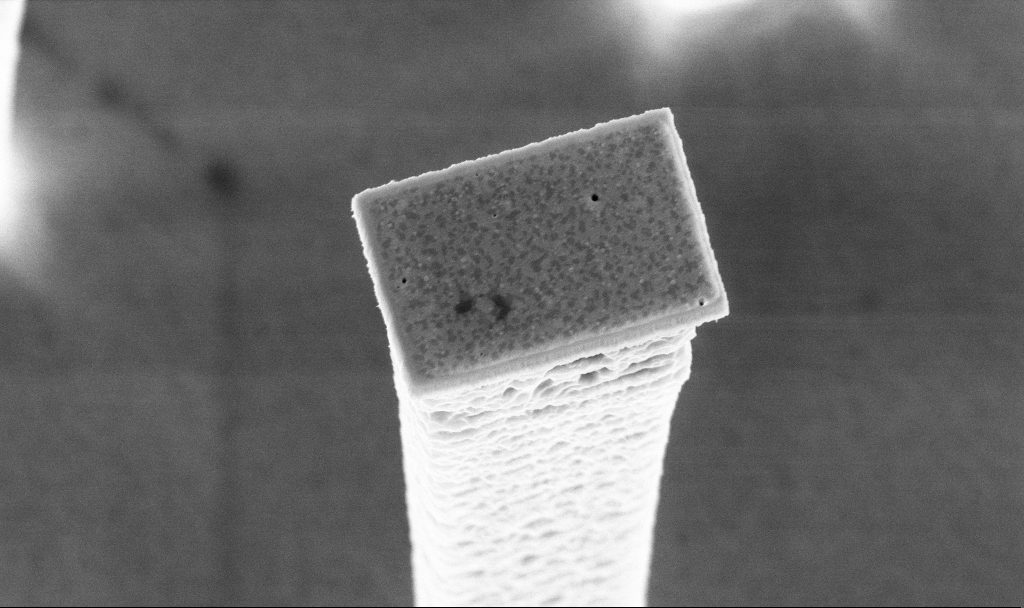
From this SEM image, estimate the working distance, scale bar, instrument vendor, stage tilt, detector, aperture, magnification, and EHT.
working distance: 3 mm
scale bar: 1000 nm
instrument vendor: Zeiss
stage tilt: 20°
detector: InLens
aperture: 30 µm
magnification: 27.28 K X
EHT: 5 kV